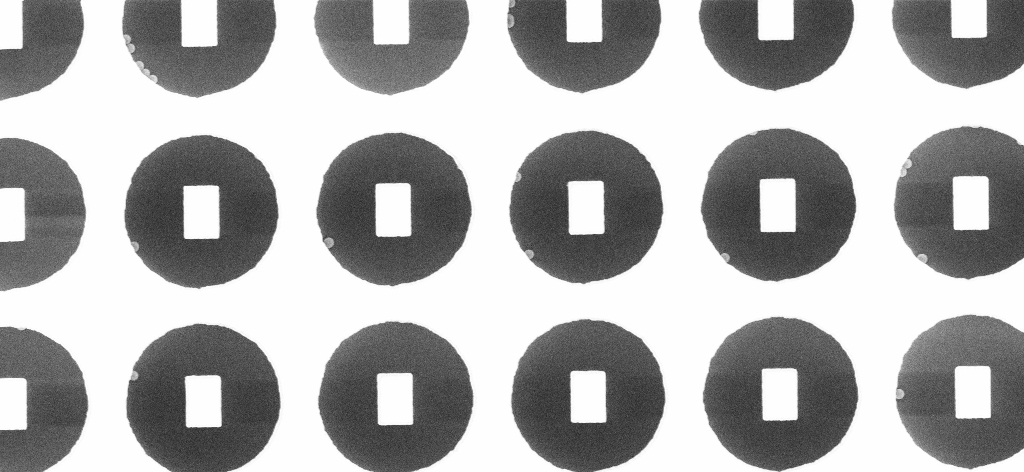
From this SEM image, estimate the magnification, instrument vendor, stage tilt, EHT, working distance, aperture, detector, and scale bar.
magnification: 6.42 K X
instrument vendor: Zeiss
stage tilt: -0°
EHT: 5 kV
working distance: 3.3 mm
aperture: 30 µm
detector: InLens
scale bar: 10000 nm